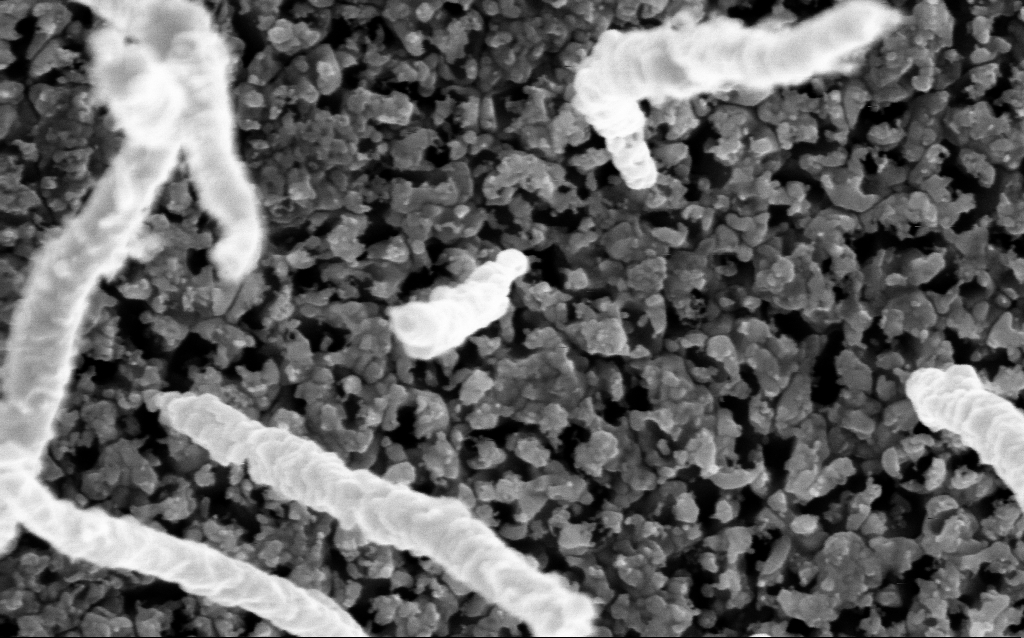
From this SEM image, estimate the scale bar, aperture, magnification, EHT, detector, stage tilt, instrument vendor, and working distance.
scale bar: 100 nm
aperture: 30 µm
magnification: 200 K X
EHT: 5 kV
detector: InLens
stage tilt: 0°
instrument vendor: Zeiss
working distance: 1.8 mm